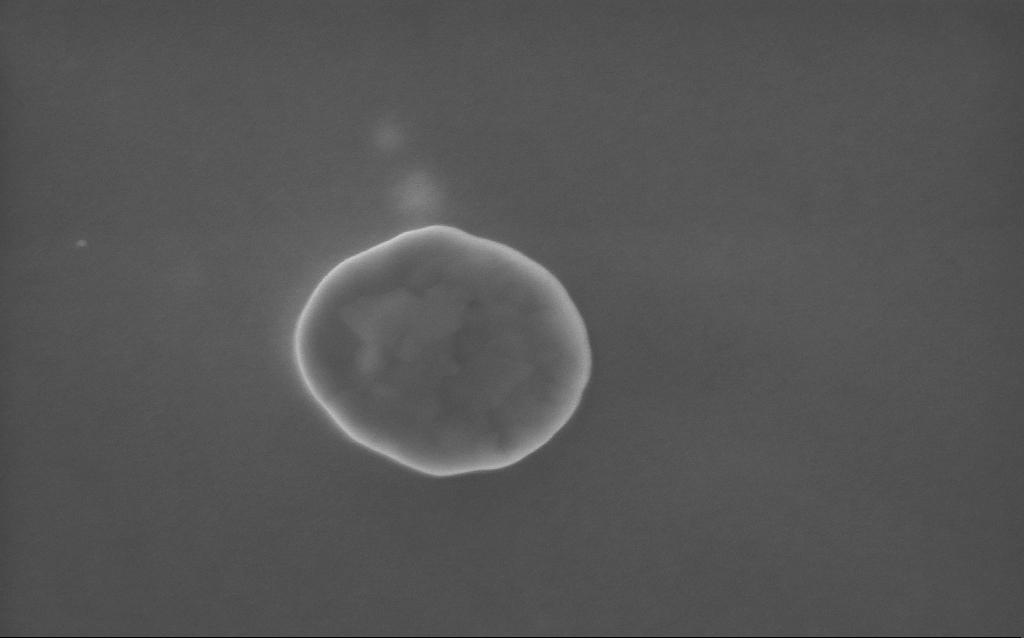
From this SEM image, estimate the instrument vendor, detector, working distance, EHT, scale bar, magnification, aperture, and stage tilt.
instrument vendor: Zeiss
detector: InLens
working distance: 3 mm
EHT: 5 kV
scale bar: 200 nm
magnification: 118.3 K X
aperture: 30 µm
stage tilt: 0°